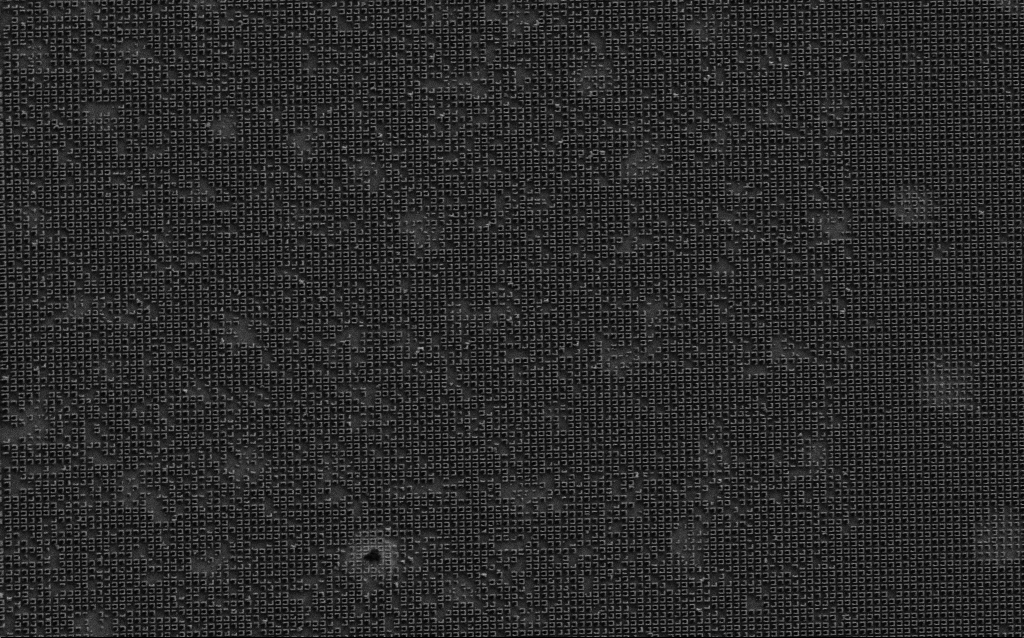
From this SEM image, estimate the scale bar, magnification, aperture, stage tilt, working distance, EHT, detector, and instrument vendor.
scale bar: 10000 nm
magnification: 5.91 K X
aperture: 30 µm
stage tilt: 0°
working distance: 6.5 mm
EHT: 3 kV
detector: SE2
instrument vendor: Zeiss